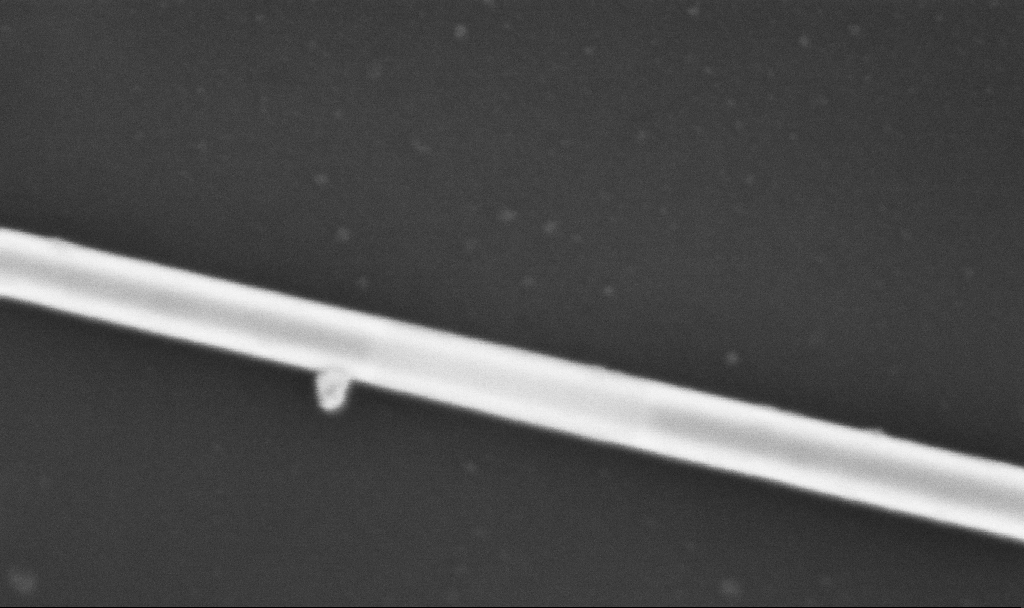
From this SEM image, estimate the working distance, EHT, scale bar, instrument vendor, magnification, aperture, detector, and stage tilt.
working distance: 6.7 mm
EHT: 5 kV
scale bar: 200 nm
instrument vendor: Zeiss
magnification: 300 K X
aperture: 30 µm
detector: InLens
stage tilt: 0°